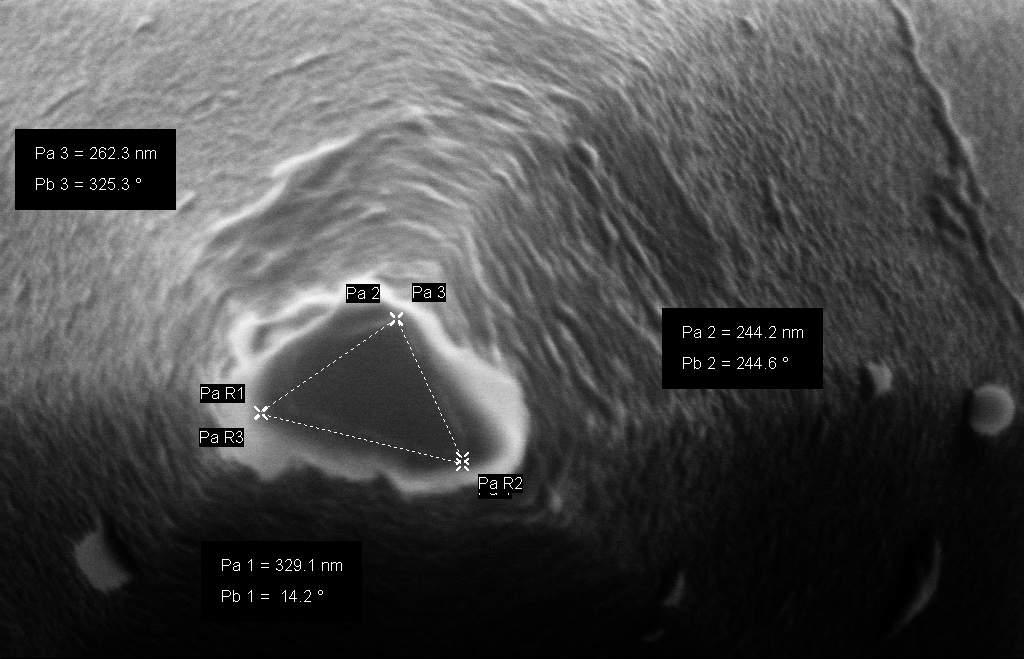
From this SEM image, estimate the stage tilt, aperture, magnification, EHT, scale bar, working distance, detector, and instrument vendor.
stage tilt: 0°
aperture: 30 µm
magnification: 231.4 K X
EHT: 10 kV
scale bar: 200 nm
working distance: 8 mm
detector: InLens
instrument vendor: Zeiss